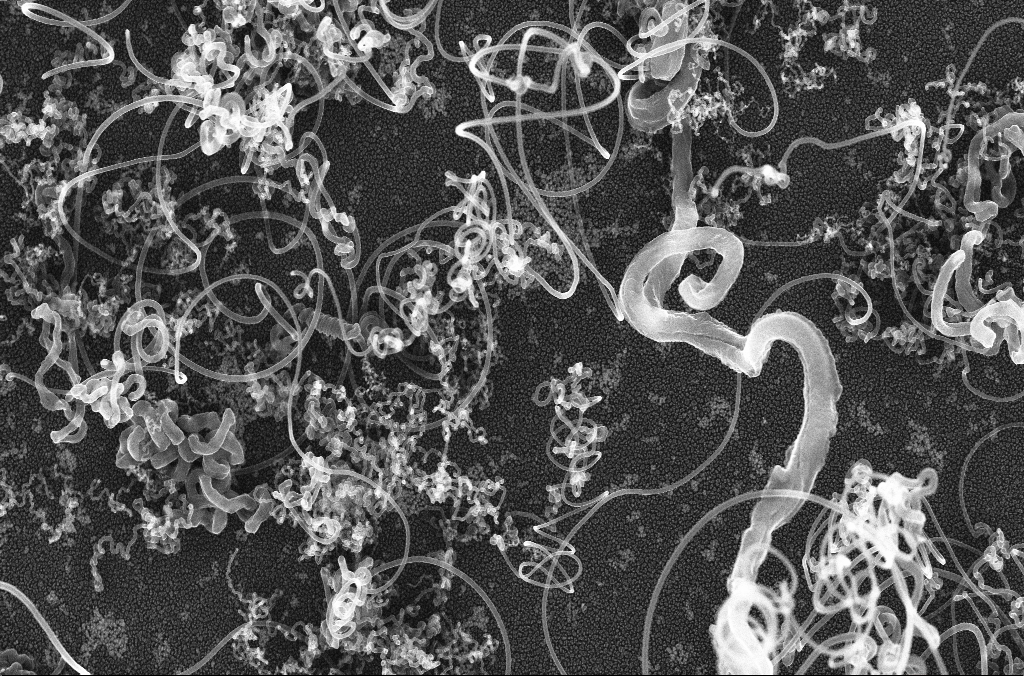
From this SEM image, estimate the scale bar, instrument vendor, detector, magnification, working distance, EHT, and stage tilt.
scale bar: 2000 nm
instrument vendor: Zeiss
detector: InLens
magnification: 15 K X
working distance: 4.2 mm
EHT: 20 kV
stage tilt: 0°